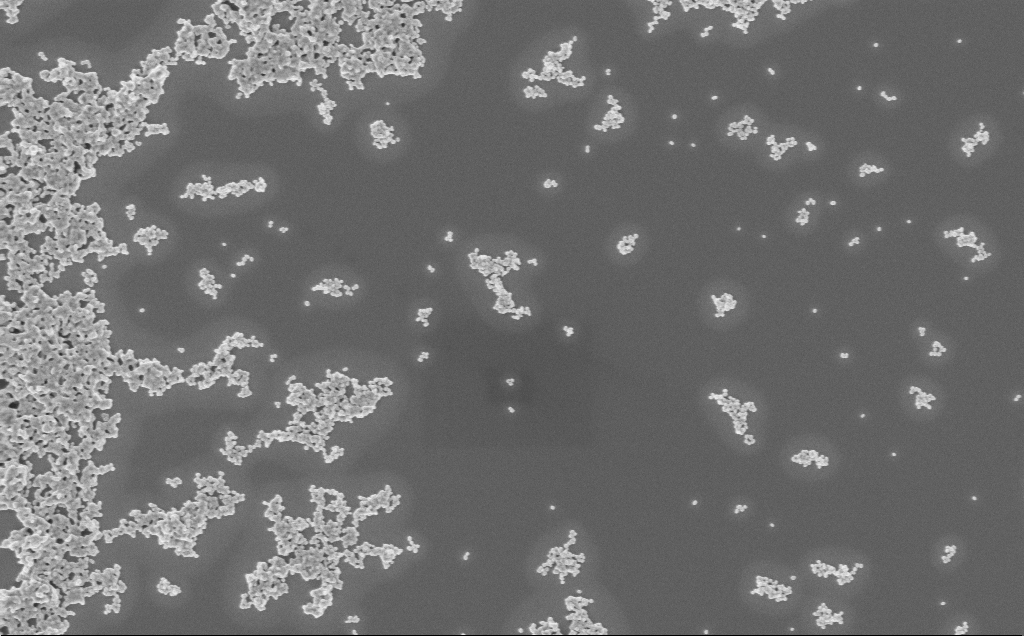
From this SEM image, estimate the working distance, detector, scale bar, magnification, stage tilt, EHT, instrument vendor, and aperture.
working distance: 5 mm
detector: InLens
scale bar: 1000 nm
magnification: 40 K X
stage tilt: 0°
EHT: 3 kV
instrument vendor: Zeiss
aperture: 30 µm